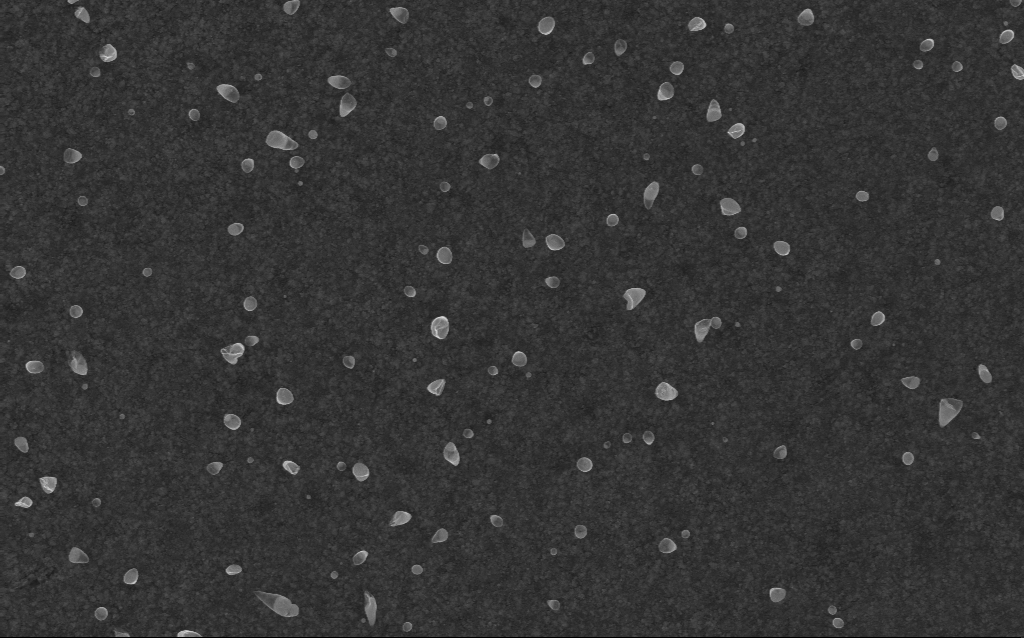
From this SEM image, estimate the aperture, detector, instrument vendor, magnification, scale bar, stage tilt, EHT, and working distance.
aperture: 30 µm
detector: InLens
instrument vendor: Zeiss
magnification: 50 K X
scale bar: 1000 nm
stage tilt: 0°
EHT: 5 kV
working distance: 2.6 mm